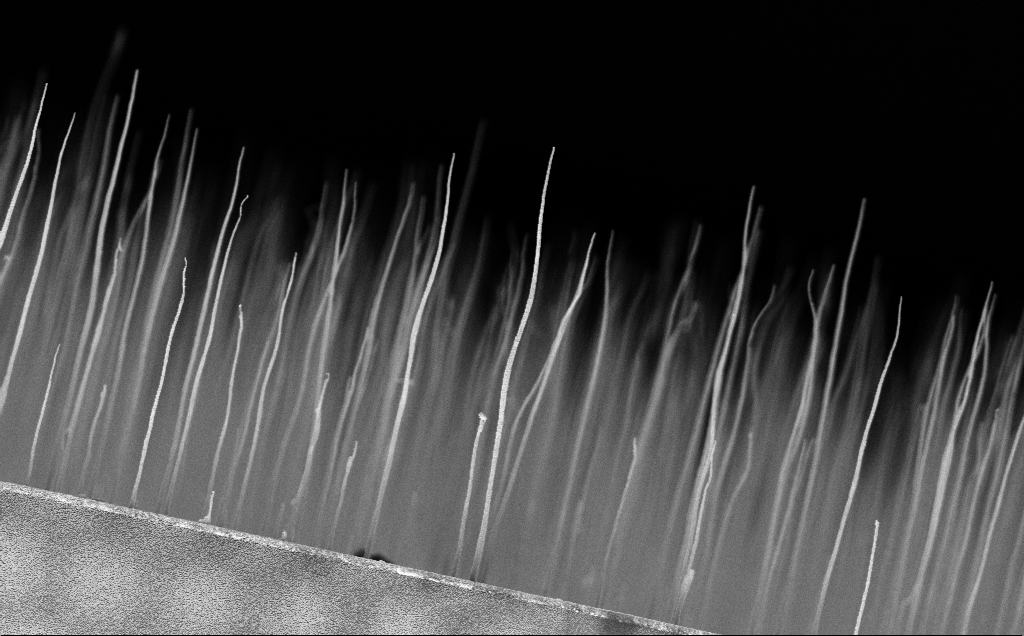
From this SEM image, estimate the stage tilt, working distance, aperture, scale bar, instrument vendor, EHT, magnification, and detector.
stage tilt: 0°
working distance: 11 mm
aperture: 30 µm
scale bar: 1000 nm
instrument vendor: Zeiss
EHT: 5 kV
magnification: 12.06 K X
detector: InLens